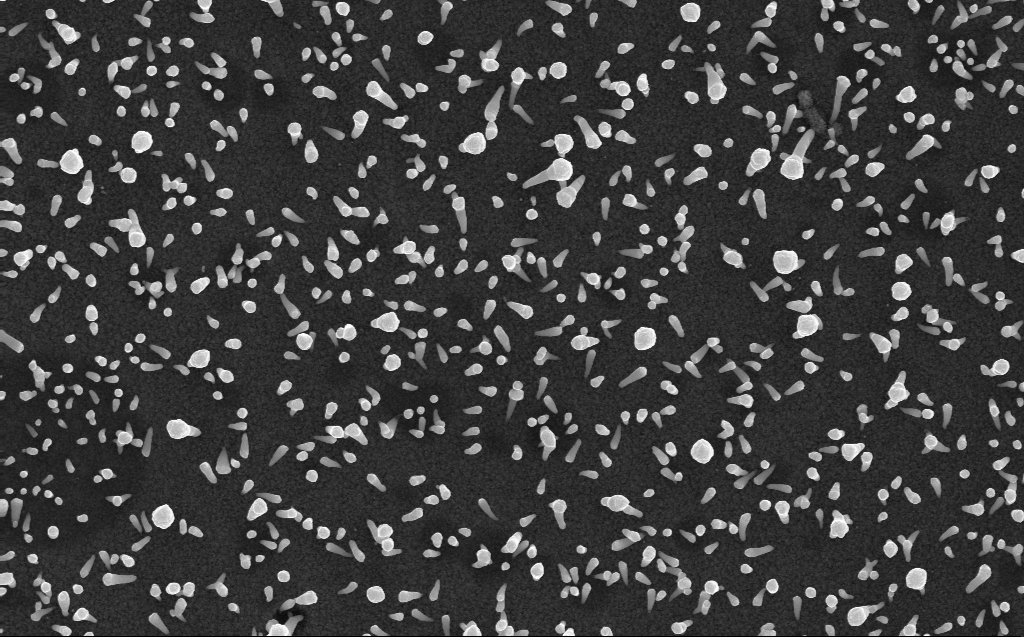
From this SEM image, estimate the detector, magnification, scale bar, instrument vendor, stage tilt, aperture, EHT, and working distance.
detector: InLens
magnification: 28.62 K X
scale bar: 1000 nm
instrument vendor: Zeiss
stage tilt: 0°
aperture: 30 µm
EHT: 10 kV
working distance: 3 mm